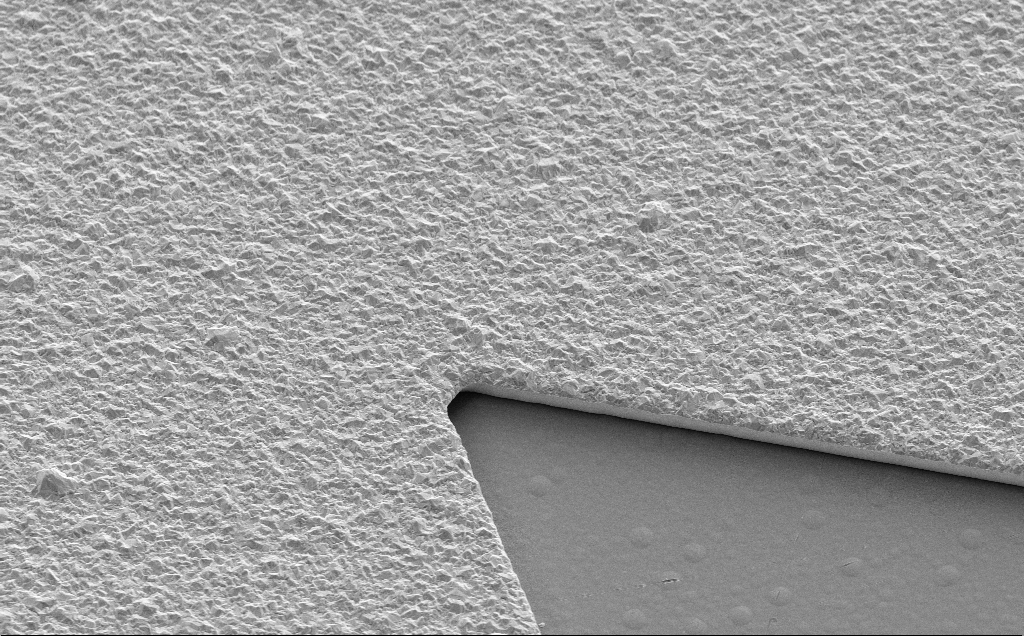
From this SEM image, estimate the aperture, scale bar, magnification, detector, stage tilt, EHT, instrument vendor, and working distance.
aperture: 30 µm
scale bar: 2000 nm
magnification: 7.78 K X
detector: SE2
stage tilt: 35°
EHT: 5 kV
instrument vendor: Zeiss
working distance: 13 mm